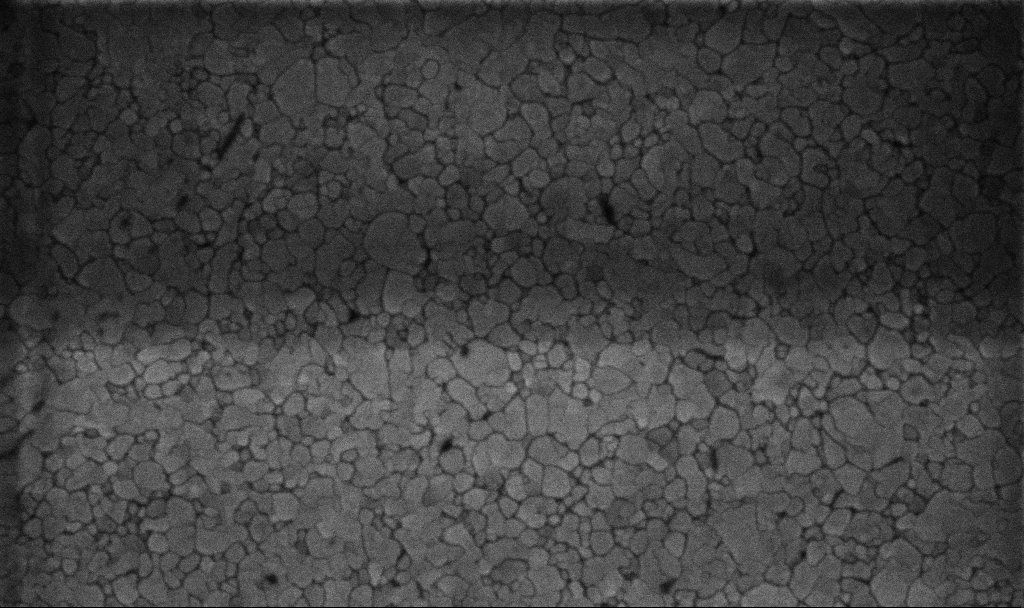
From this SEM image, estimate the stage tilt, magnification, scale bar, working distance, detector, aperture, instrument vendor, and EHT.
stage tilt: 0°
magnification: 100.15 K X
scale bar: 200 nm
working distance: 3.1 mm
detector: InLens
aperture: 30 µm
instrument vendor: Zeiss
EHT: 5 kV